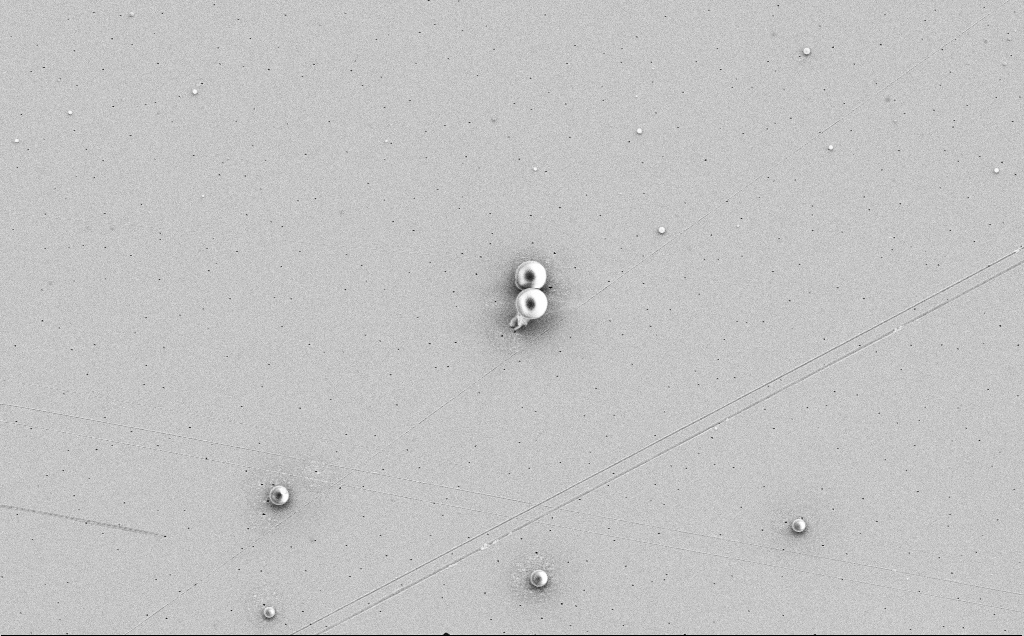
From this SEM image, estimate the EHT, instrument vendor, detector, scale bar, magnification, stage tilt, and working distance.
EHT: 5 kV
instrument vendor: Zeiss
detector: SE2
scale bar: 10000 nm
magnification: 4.2 K X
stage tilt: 0°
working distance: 12 mm